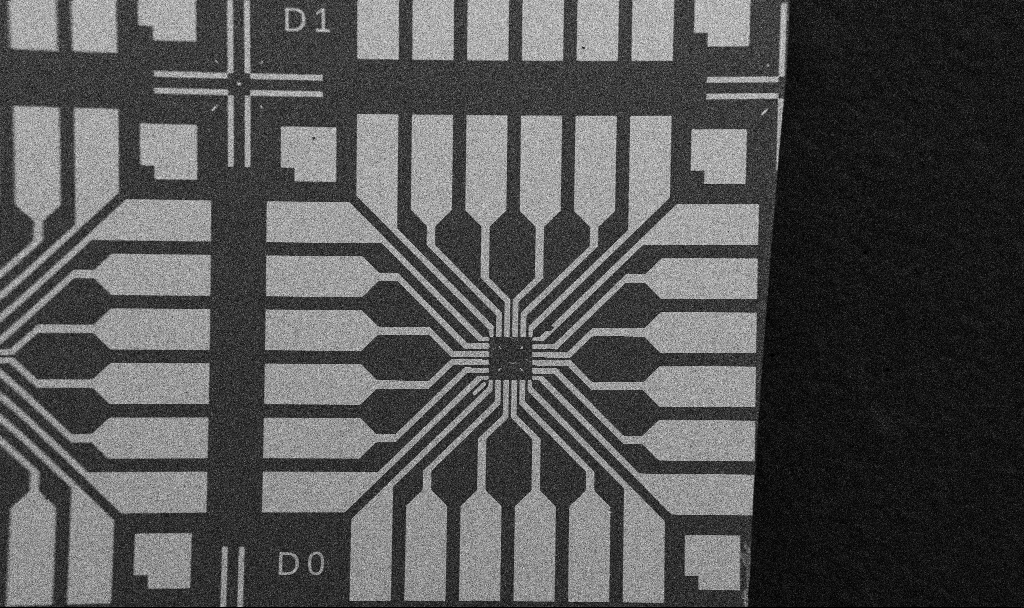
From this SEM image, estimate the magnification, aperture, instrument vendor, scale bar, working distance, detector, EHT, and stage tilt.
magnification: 0.1 K X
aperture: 30 µm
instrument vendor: Zeiss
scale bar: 200000 nm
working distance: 10.7 mm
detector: SE2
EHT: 5 kV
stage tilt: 0°